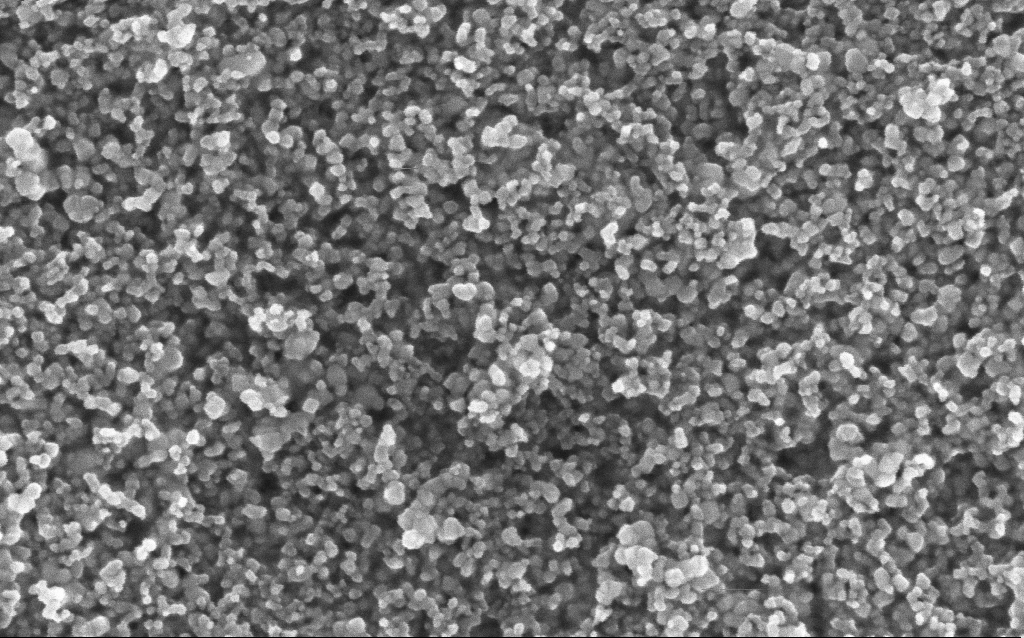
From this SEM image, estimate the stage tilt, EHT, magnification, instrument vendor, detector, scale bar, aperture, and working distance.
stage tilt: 0°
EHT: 5 kV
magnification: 162.39 K X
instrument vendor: Zeiss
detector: InLens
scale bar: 100 nm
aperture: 30 µm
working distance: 6.4 mm